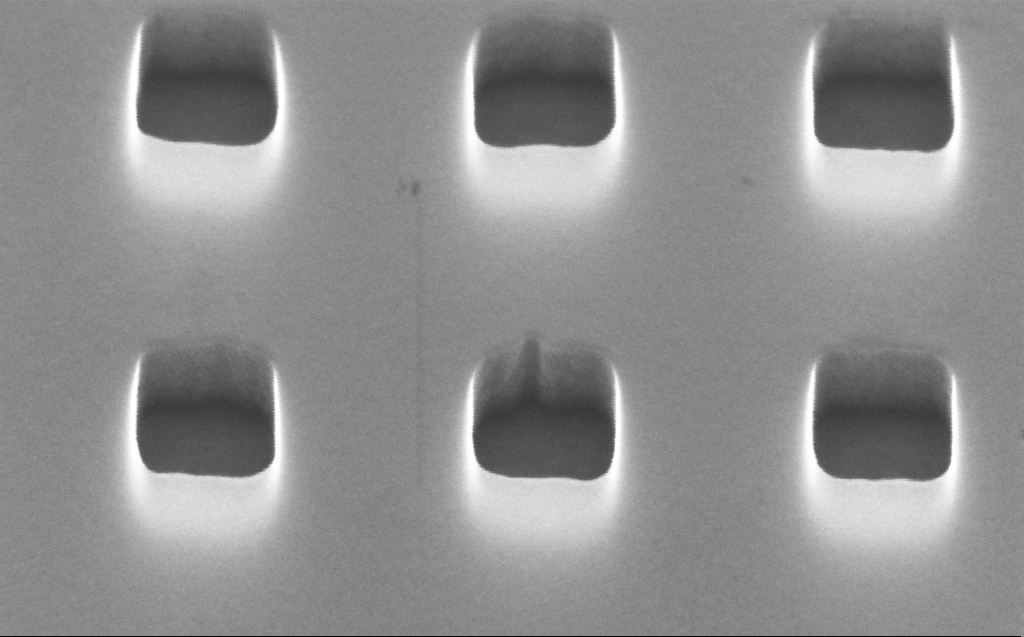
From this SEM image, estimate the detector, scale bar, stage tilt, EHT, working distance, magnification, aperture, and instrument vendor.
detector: InLens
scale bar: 200 nm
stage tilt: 45°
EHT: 10 kV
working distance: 3 mm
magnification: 251.48 K X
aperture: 30 µm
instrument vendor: Zeiss